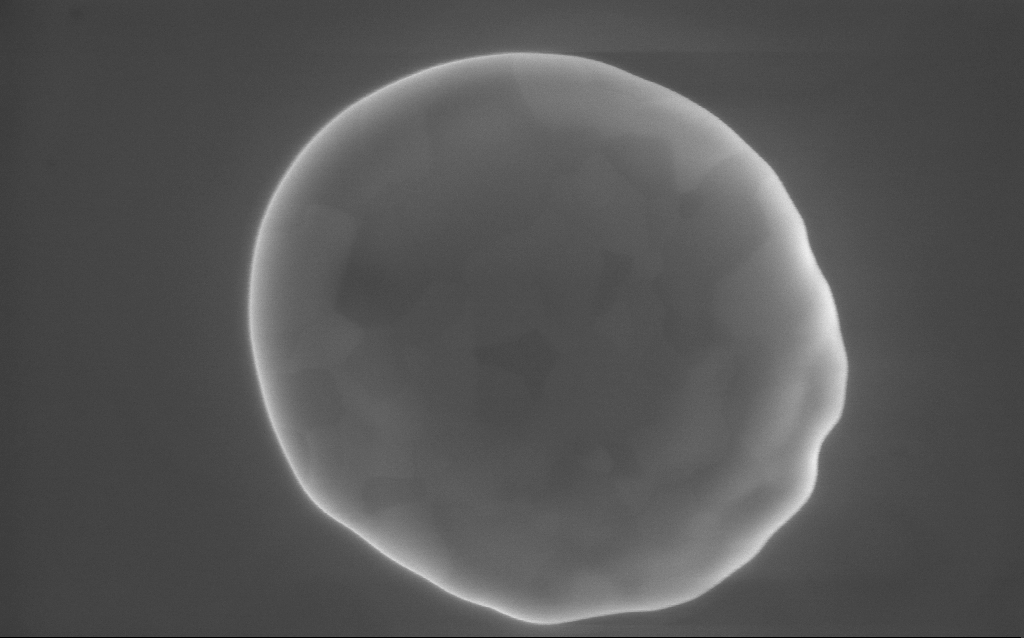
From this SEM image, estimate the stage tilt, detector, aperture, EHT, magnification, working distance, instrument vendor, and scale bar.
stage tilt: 0°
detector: InLens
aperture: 30 µm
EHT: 10 kV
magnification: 90.94 K X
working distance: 2 mm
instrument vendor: Zeiss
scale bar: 200 nm